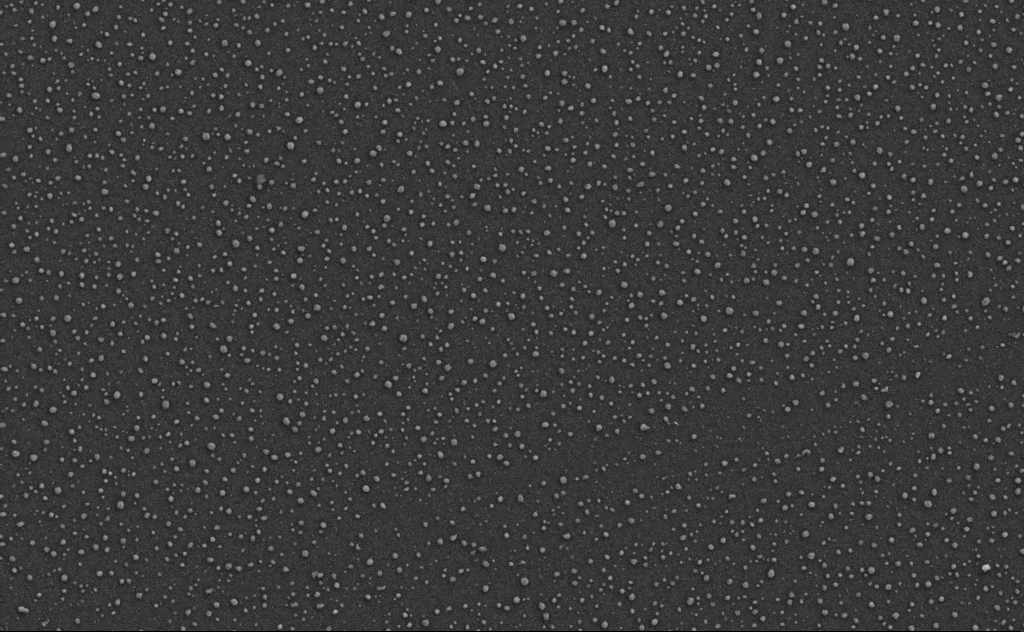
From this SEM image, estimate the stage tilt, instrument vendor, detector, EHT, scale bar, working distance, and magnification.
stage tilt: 0°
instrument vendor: Zeiss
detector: SE2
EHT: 5 kV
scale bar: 2000 nm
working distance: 6 mm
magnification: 20 K X